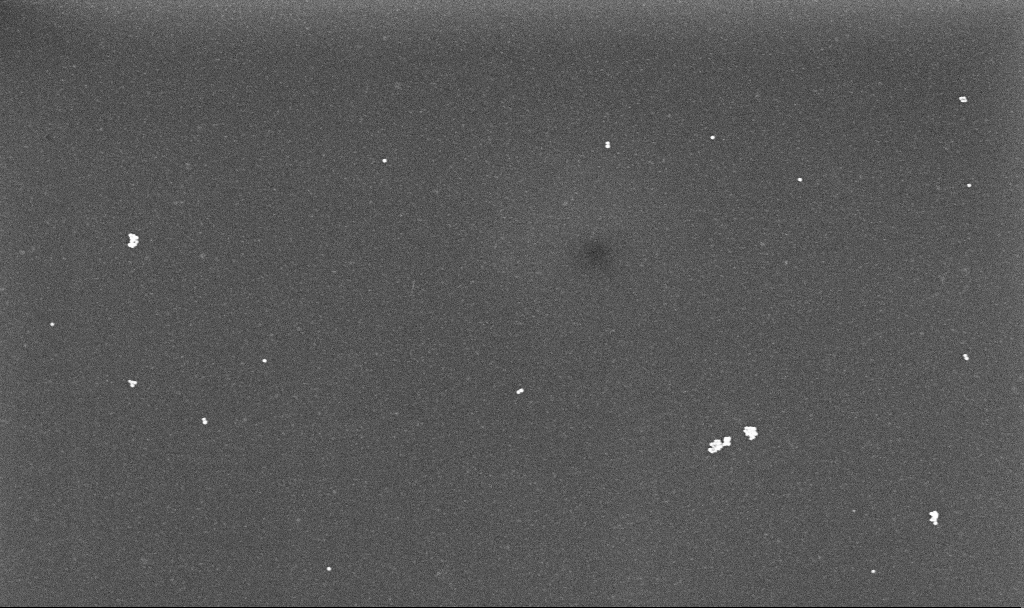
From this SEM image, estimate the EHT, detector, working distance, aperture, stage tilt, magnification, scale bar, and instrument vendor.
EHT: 10 kV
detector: InLens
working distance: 3.1 mm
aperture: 30 µm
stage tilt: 0°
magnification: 48.56 K X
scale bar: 1000 nm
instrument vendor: Zeiss